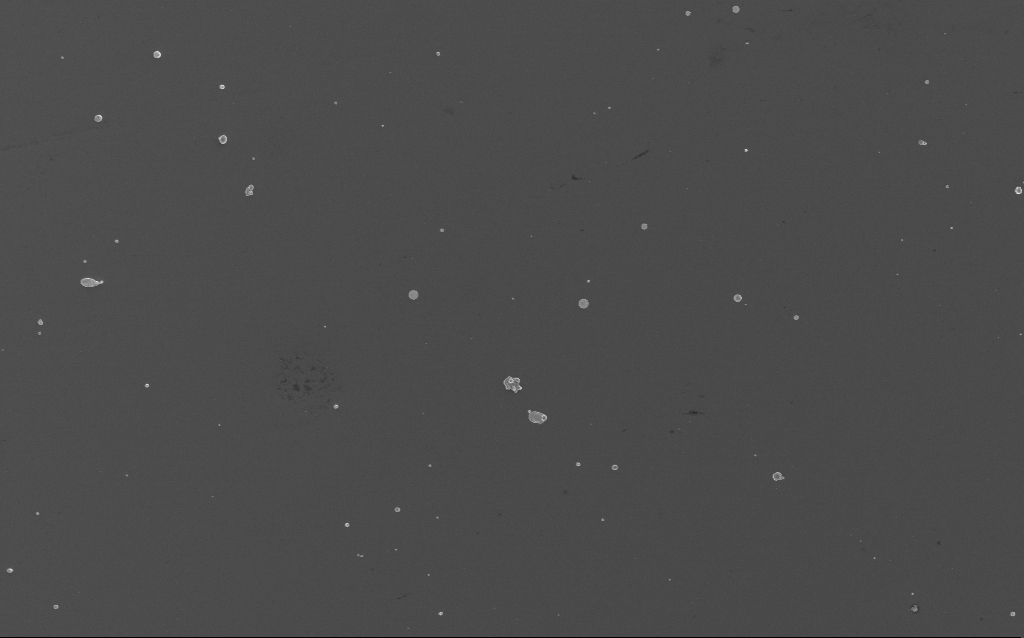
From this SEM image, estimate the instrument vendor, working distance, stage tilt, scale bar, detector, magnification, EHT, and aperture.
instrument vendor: Zeiss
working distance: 4 mm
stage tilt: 0°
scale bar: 10000 nm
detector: InLens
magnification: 1.88 K X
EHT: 3 kV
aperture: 30 µm